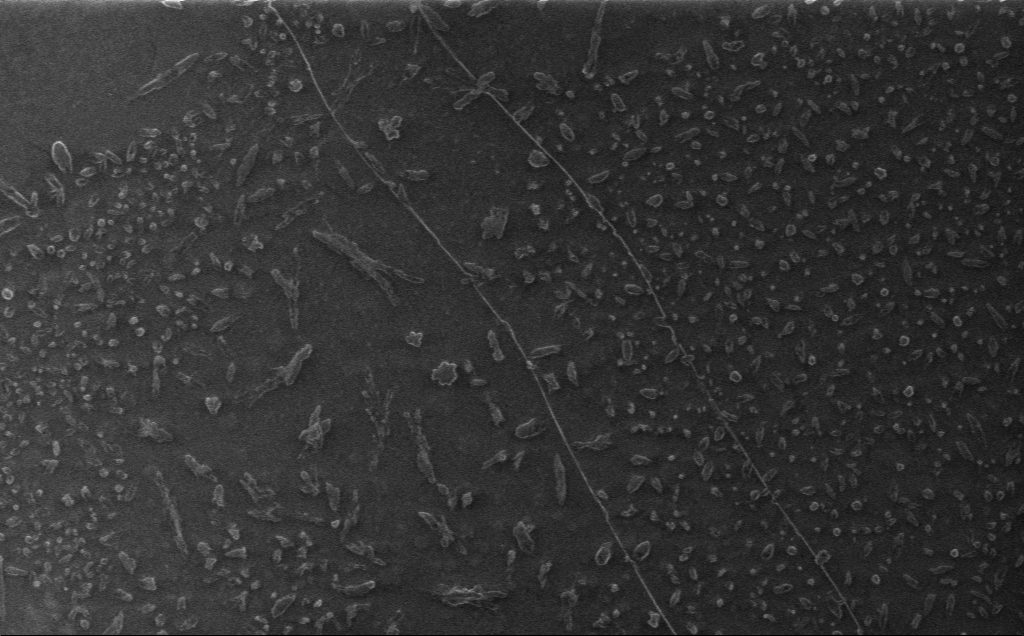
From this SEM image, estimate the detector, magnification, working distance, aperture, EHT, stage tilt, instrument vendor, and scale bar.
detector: InLens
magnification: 3.37 K X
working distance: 3 mm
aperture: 30 µm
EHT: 1 kV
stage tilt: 0°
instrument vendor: Zeiss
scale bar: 20000 nm